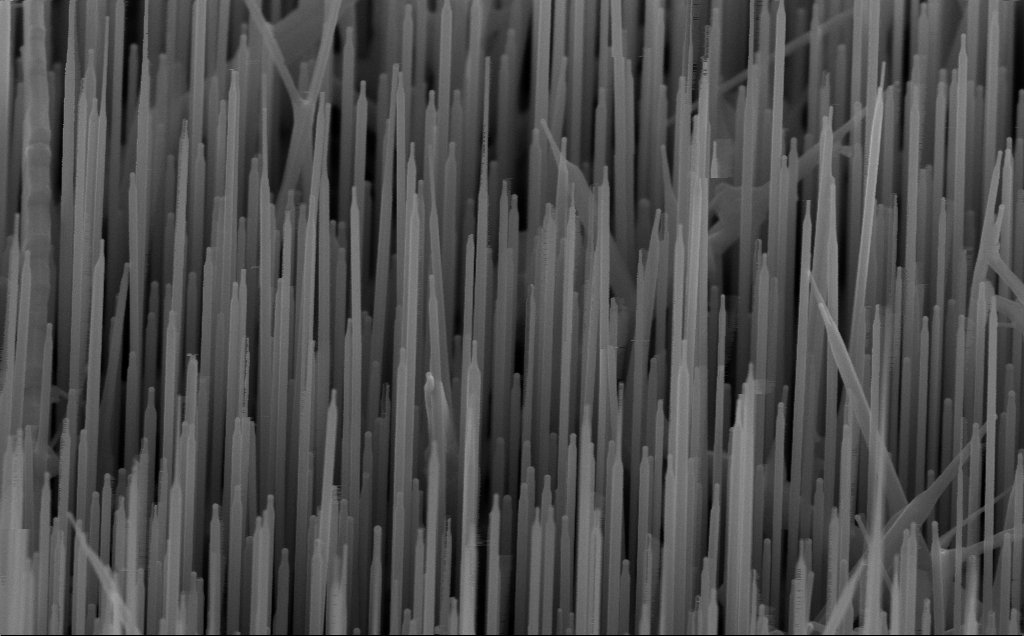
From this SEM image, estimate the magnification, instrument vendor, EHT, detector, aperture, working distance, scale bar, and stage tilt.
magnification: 80 K X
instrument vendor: Zeiss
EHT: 10 kV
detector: InLens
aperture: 30 µm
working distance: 7 mm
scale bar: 200 nm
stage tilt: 45°